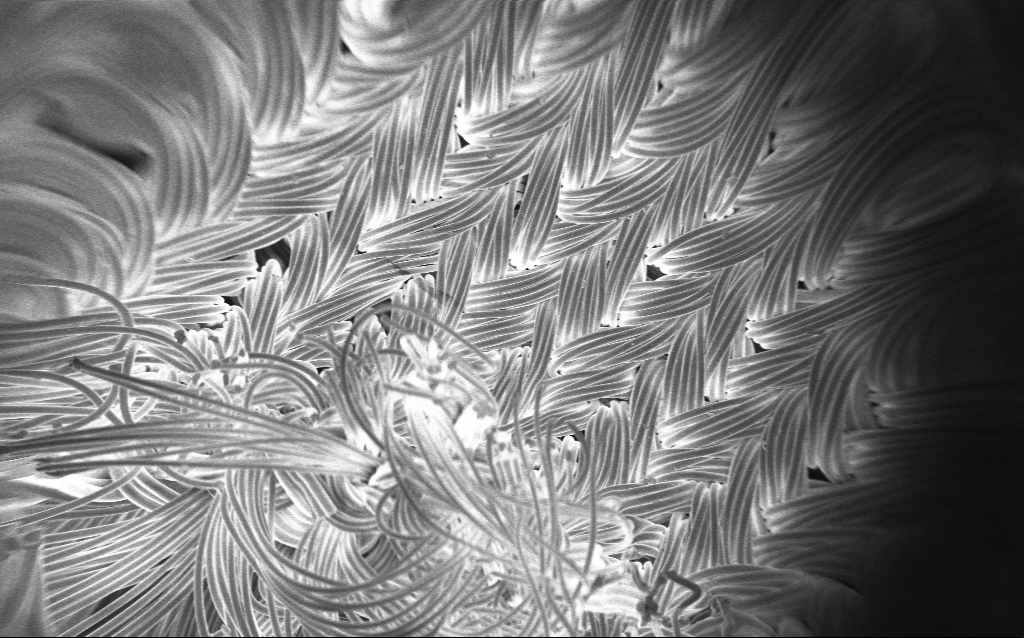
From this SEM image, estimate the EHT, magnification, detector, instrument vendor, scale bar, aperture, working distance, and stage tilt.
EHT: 1 kV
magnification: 0.121 K X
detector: InLens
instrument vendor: Zeiss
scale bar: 100000 nm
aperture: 30 µm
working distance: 4 mm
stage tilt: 0°